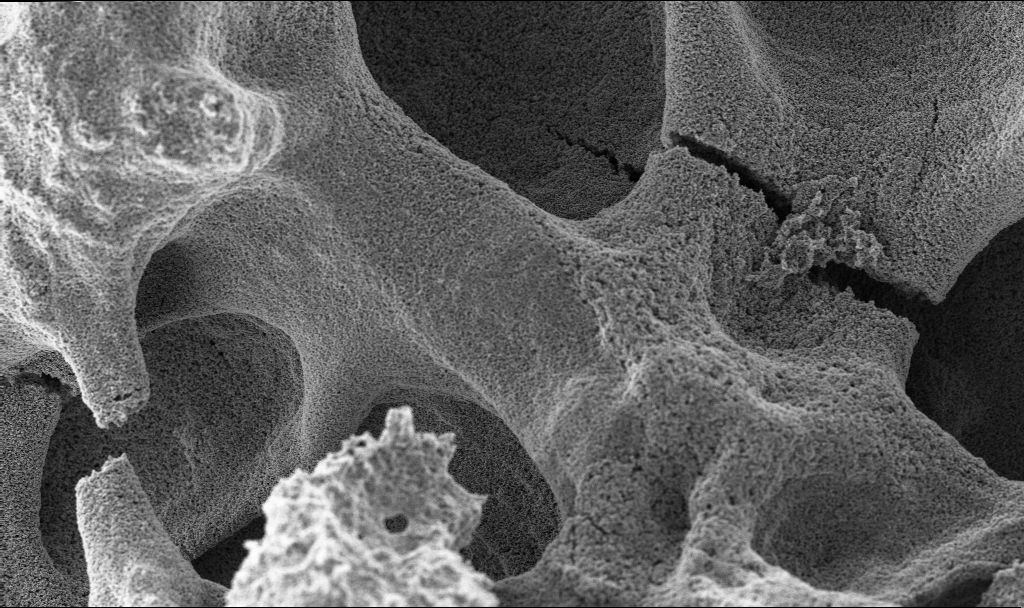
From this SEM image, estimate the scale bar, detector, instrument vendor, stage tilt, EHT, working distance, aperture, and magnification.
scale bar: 10000 nm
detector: InLens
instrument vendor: Zeiss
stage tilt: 0°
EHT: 3 kV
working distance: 2.4 mm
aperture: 30 µm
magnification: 6.9 K X